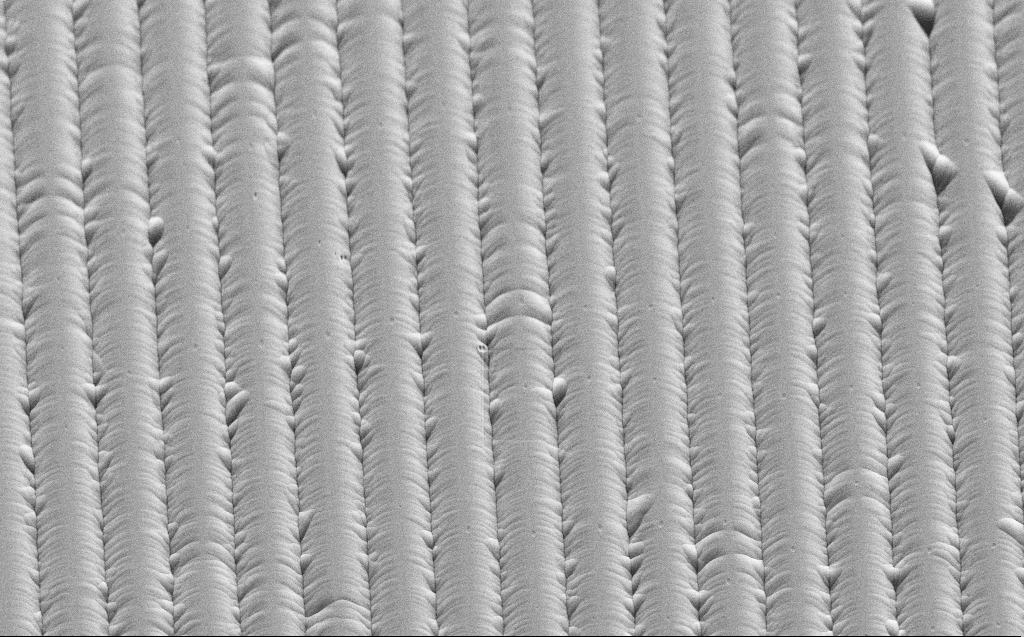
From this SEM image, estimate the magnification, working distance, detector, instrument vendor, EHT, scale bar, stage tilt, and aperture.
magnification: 2.41 K X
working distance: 9 mm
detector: SE2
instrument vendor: Zeiss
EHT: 3 kV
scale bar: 10000 nm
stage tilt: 45°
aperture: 30 µm